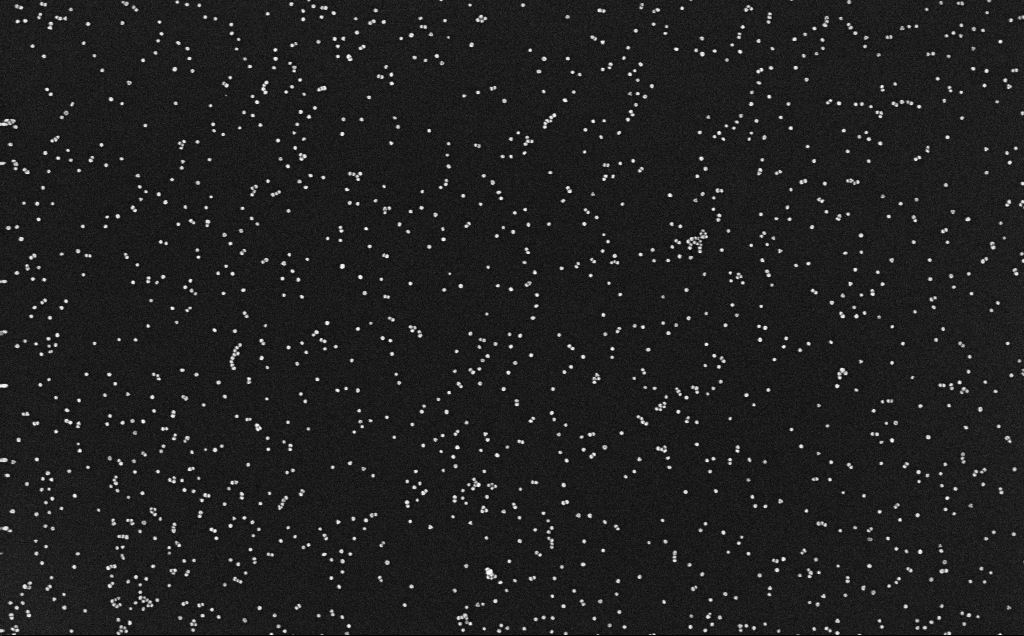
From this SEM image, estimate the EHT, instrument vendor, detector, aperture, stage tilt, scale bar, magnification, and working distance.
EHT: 10 kV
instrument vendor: Zeiss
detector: InLens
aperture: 30 µm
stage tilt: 0°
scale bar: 200 nm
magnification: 100 K X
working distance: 3.1 mm